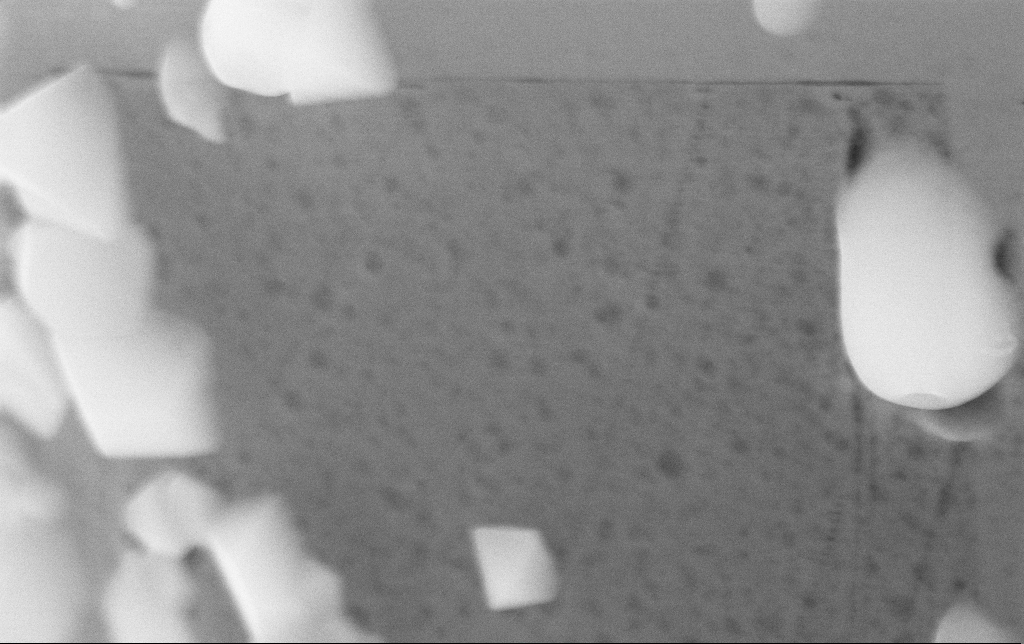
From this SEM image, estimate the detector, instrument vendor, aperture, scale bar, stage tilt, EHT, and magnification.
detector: InLens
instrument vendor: Zeiss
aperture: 30 µm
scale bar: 1000 nm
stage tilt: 0°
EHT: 15 kV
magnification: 60.09 K X